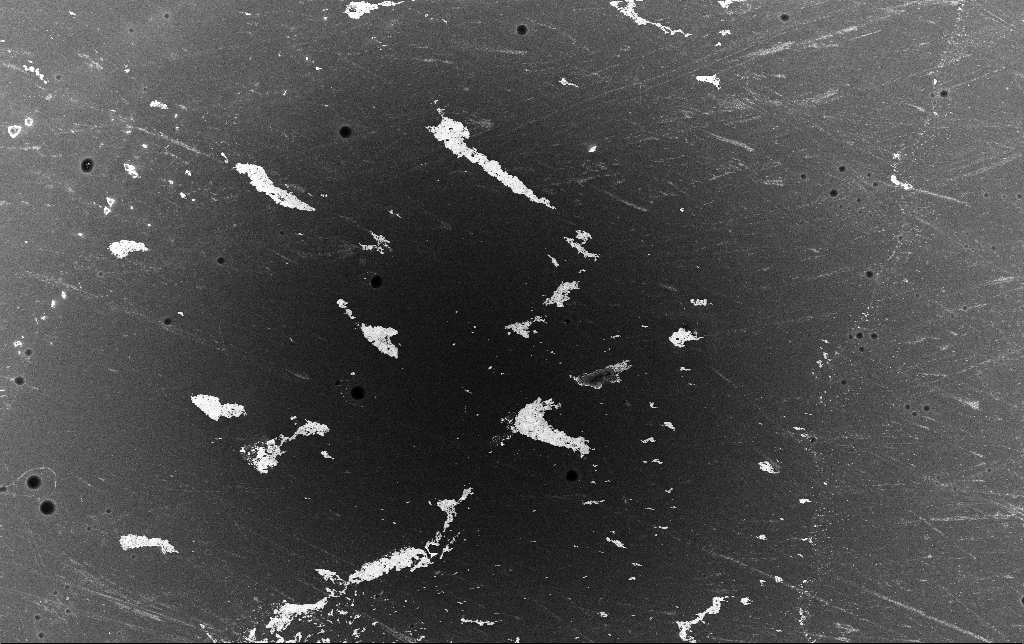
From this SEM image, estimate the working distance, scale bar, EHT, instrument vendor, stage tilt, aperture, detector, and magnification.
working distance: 3.4 mm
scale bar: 100000 nm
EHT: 10 kV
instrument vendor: Zeiss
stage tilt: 0°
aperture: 30 µm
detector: InLens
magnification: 0.523 K X